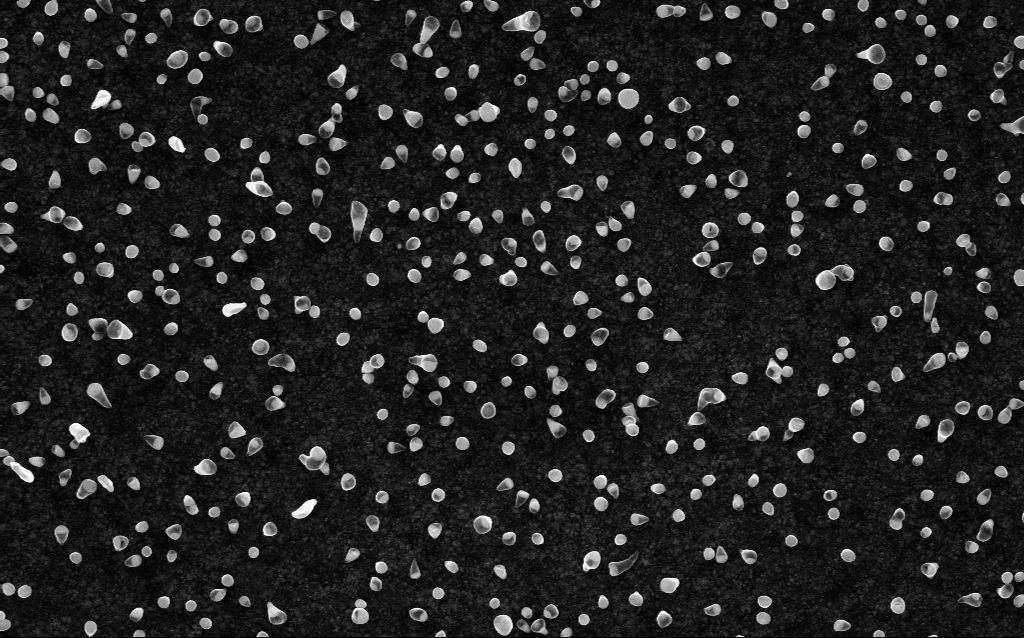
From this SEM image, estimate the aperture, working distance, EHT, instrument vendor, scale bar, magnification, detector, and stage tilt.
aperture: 30 µm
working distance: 1.9 mm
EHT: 5 kV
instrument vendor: Zeiss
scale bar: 1000 nm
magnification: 50 K X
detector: InLens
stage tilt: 0°